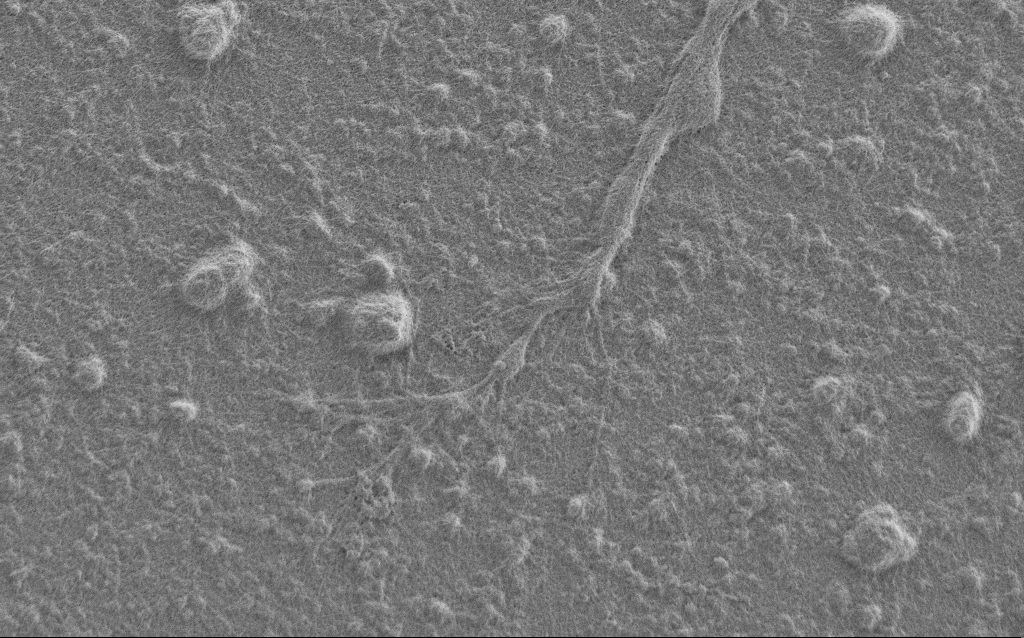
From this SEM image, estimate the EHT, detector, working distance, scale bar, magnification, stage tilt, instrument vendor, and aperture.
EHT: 1 kV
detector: SE2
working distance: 6 mm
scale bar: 2000 nm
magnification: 7.5 K X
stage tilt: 0°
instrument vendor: Zeiss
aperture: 30 µm